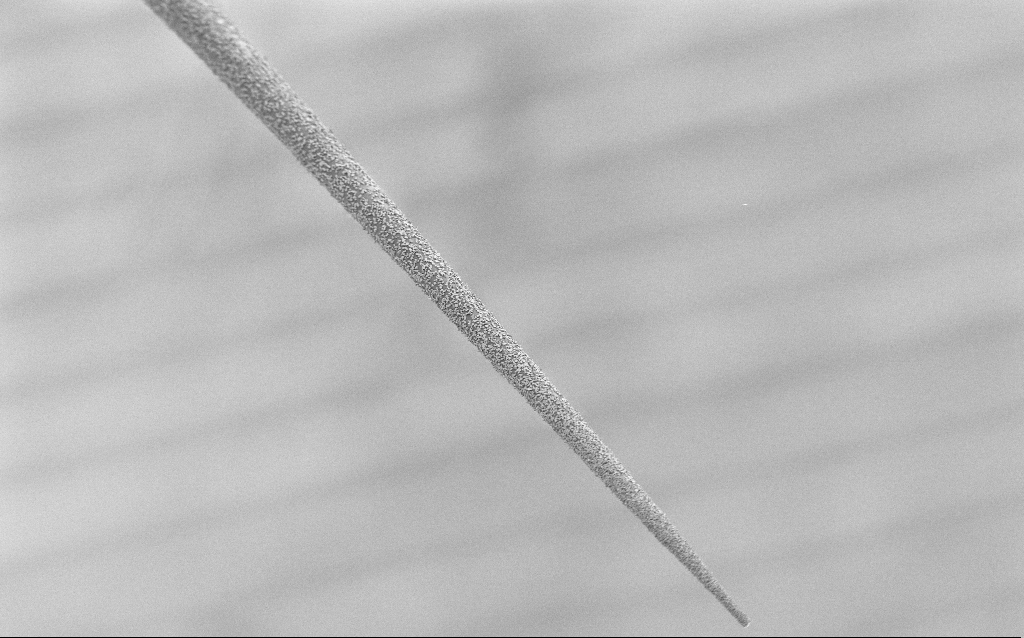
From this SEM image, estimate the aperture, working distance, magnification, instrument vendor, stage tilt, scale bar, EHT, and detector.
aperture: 30 µm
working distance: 7.9 mm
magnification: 0.5 K X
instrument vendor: Zeiss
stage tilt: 45°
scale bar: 100000 nm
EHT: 1.5 kV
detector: SE2